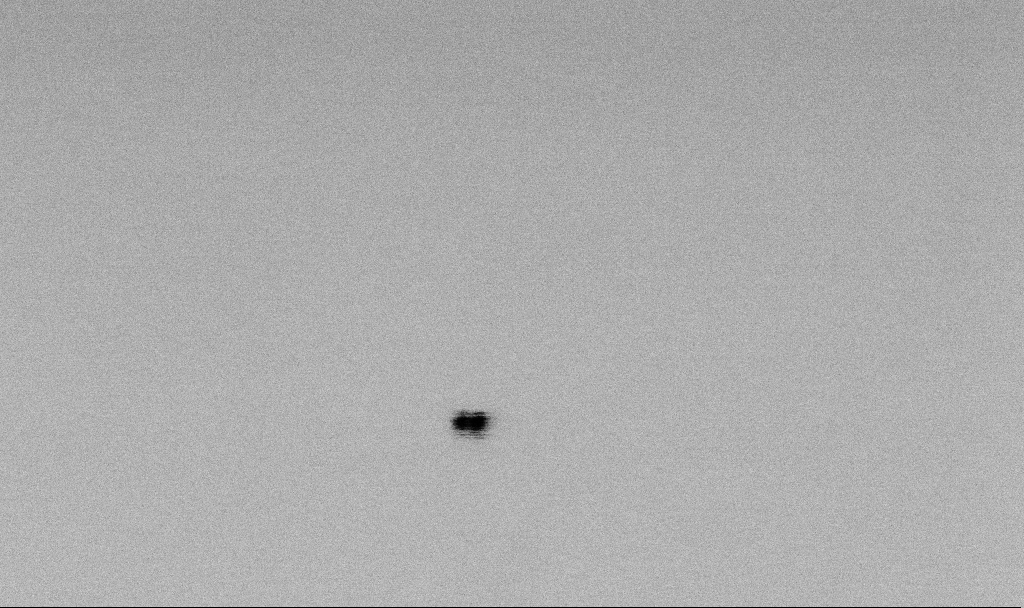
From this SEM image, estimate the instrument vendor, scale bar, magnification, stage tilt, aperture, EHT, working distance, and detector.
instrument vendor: Zeiss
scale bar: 100 nm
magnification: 316.68 K X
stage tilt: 0°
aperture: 30 µm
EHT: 2 kV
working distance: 6.5 mm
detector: SE2